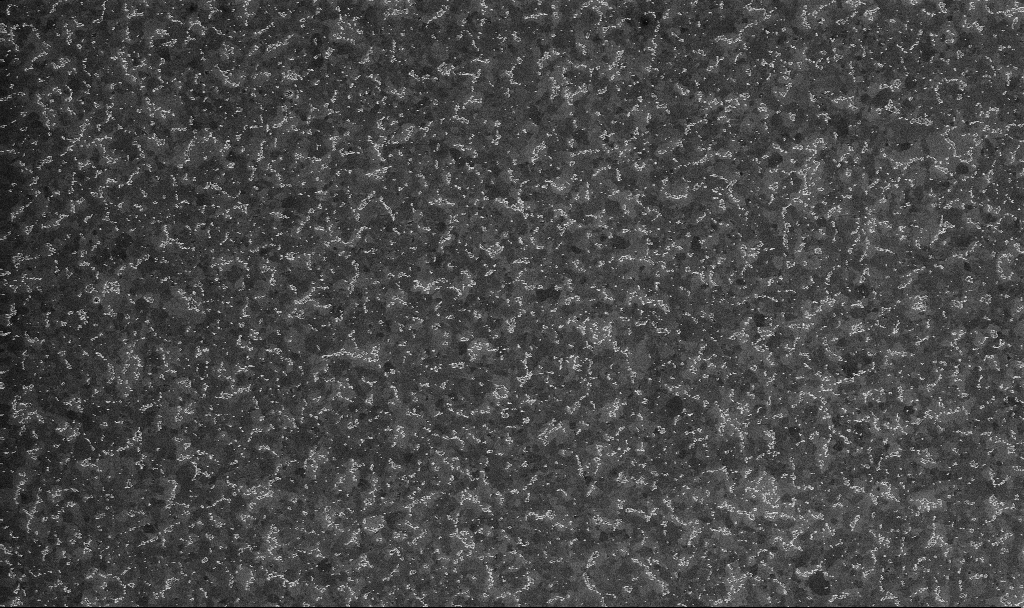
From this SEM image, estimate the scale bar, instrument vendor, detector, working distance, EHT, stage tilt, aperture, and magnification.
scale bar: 1000 nm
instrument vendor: Zeiss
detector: InLens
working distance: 3.3 mm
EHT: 10 kV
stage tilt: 0°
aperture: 30 µm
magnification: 20 K X